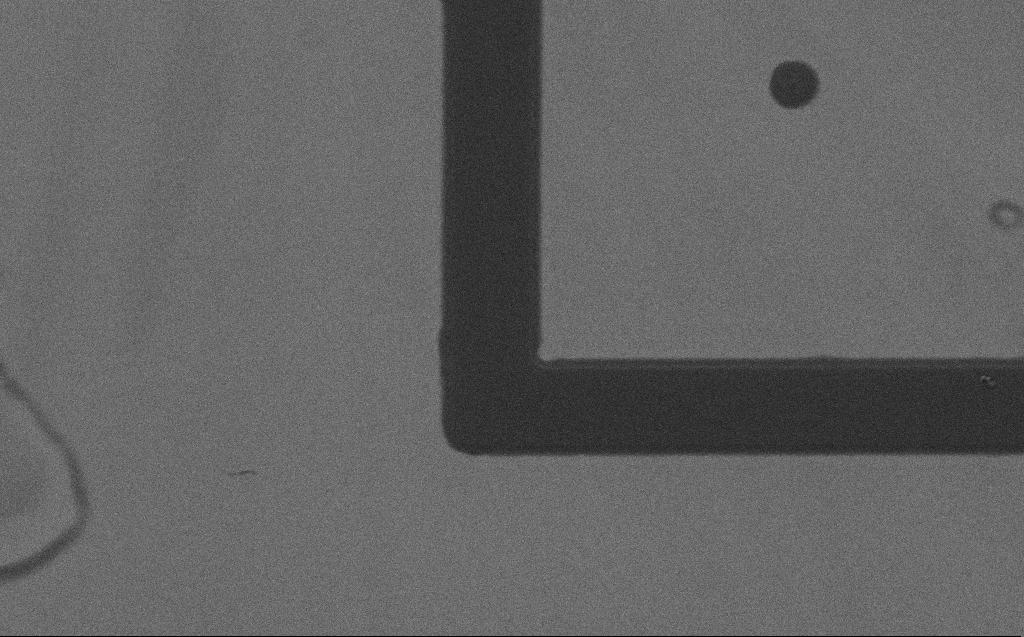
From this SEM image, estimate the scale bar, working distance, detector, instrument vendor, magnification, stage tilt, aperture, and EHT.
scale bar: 10000 nm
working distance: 4 mm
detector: SE2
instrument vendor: Zeiss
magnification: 2.52 K X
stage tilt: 0°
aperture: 30 µm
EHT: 1.2 kV